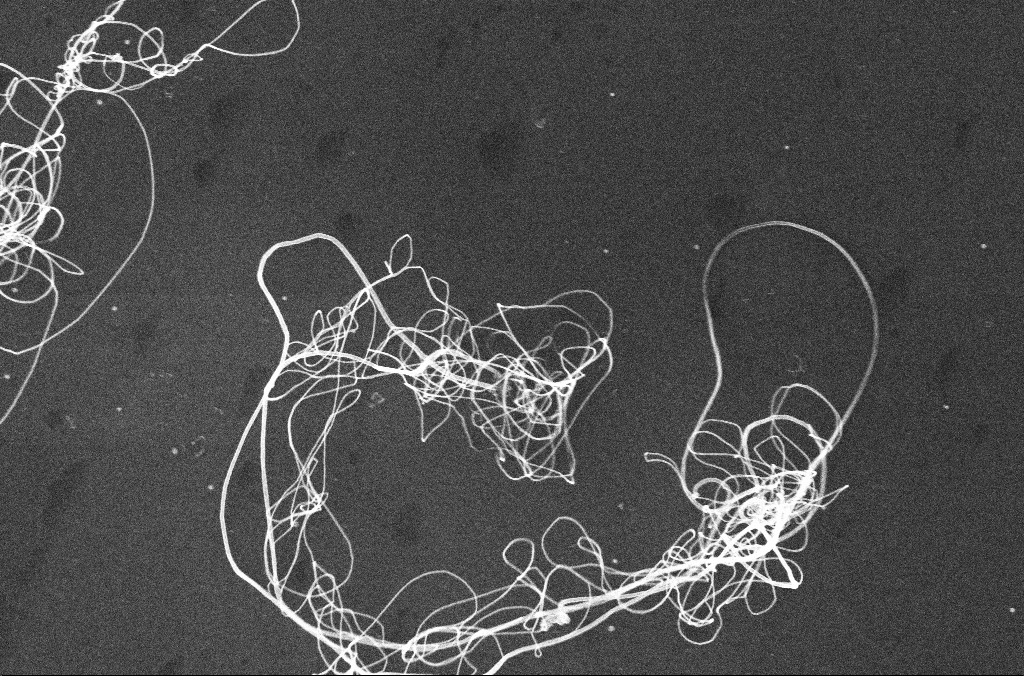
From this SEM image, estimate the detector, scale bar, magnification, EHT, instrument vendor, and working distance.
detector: InLens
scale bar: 1000 nm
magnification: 50 K X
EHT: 10 kV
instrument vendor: Zeiss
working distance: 3.3 mm